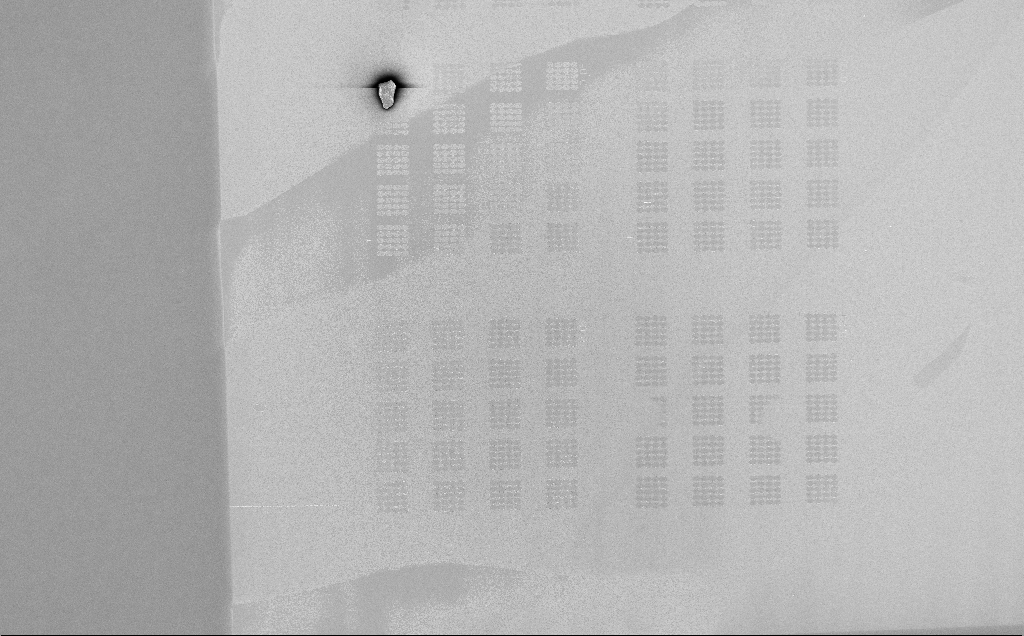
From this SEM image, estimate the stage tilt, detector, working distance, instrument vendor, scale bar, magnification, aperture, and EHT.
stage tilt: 0°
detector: InLens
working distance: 6 mm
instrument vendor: Zeiss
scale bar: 100000 nm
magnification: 0.3 K X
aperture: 30 µm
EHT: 5 kV